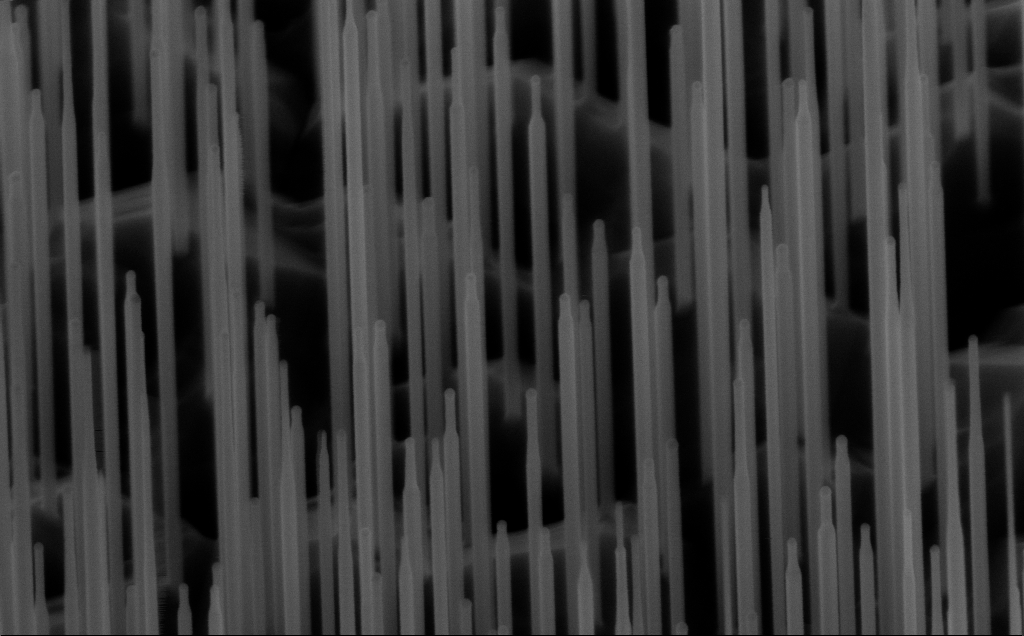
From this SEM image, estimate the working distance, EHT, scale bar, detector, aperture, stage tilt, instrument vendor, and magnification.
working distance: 6 mm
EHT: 10 kV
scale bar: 200 nm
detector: InLens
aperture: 30 µm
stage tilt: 45°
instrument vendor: Zeiss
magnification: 80 K X